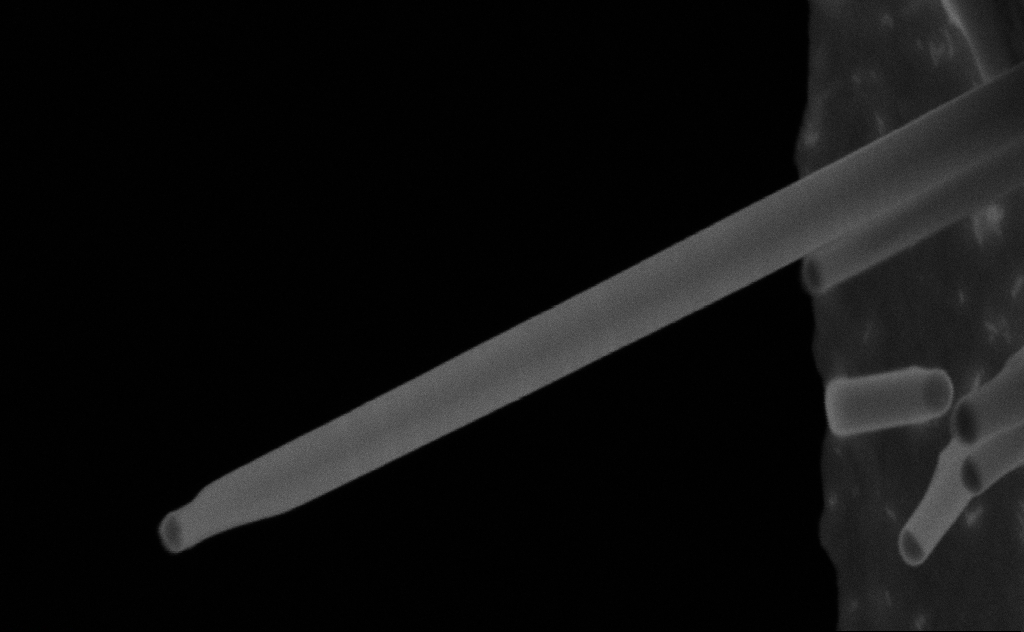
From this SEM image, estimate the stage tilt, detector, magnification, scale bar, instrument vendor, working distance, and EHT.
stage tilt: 0°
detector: SE2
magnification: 222.97 K X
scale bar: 200 nm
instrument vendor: Zeiss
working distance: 9 mm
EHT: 20 kV